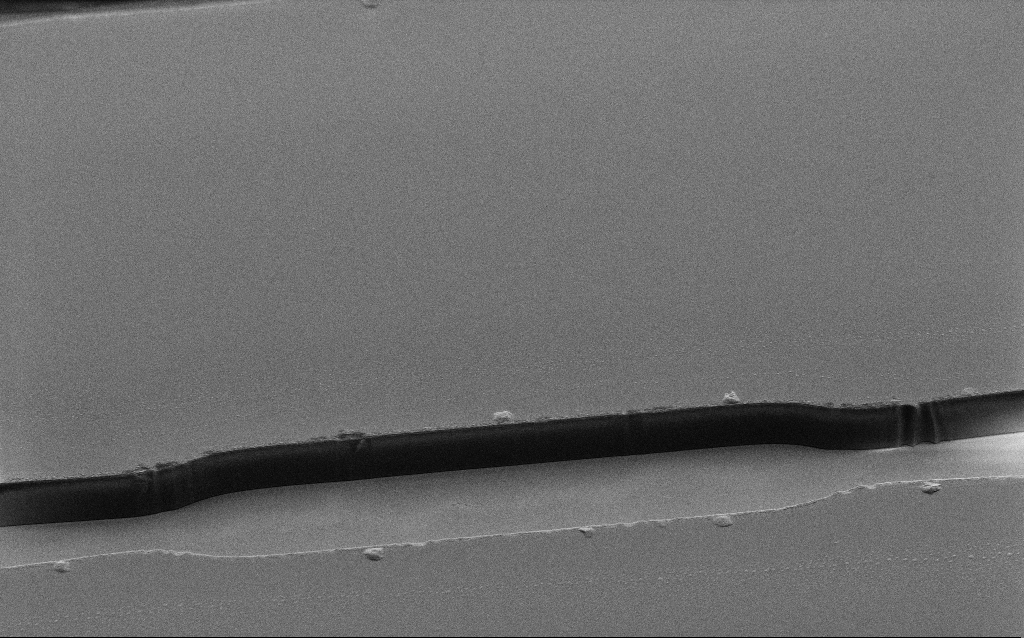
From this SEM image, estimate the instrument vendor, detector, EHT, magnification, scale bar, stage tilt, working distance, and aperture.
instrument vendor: Zeiss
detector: SE2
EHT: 1.2 kV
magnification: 2.6 K X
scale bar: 20000 nm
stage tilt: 45°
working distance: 6 mm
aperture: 30 µm